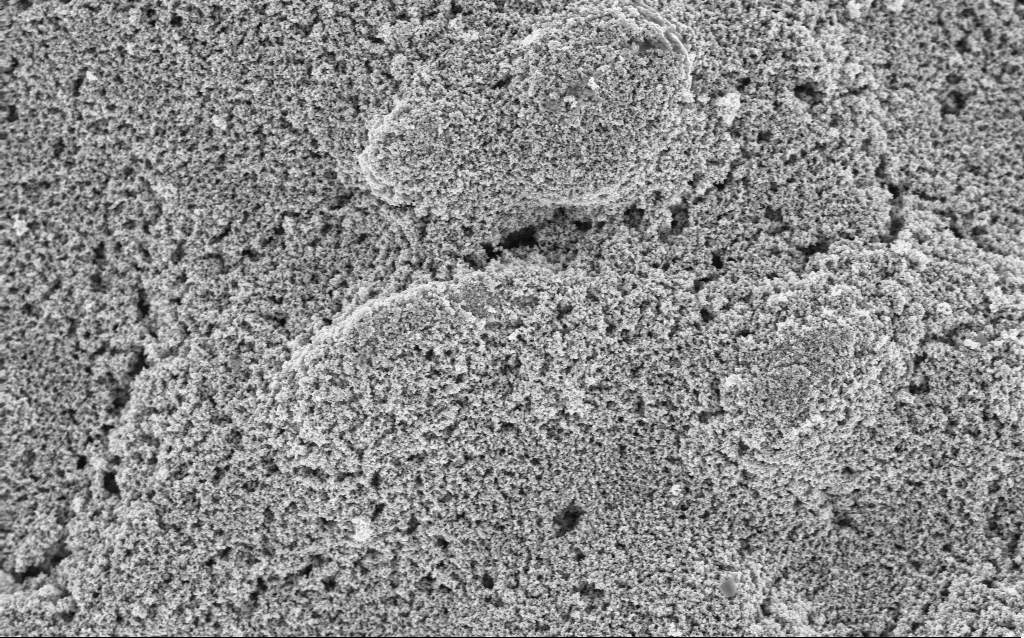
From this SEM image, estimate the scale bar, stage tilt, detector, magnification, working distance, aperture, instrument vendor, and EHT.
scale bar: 1000 nm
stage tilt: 0°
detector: InLens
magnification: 23.9 K X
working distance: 7.6 mm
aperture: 30 µm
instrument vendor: Zeiss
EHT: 3 kV